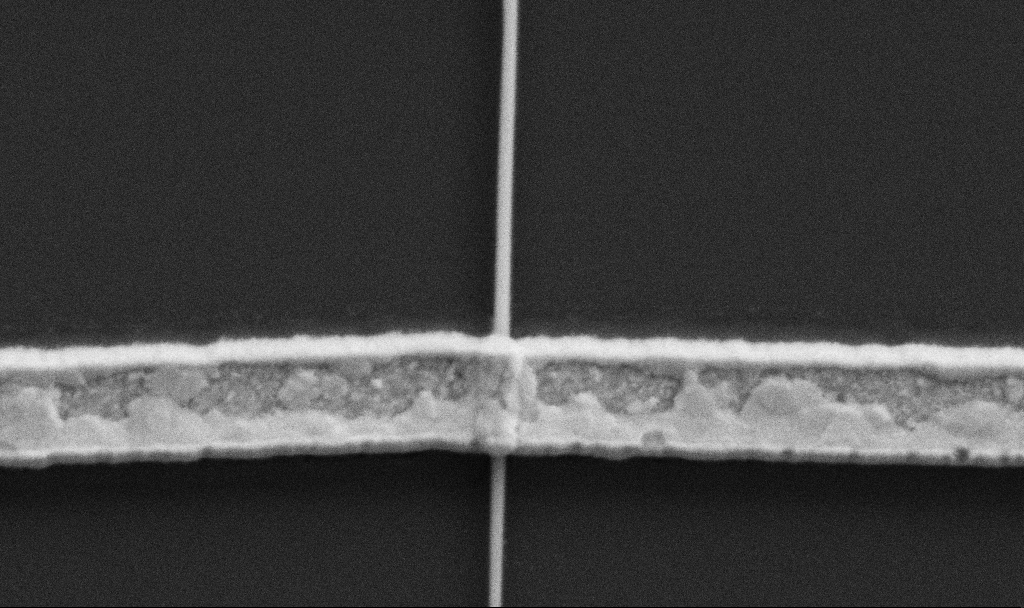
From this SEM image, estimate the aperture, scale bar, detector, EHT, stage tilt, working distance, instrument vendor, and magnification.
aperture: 30 µm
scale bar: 1000 nm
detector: SE2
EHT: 5 kV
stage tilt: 0°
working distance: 10.8 mm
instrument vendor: Zeiss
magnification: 60 K X